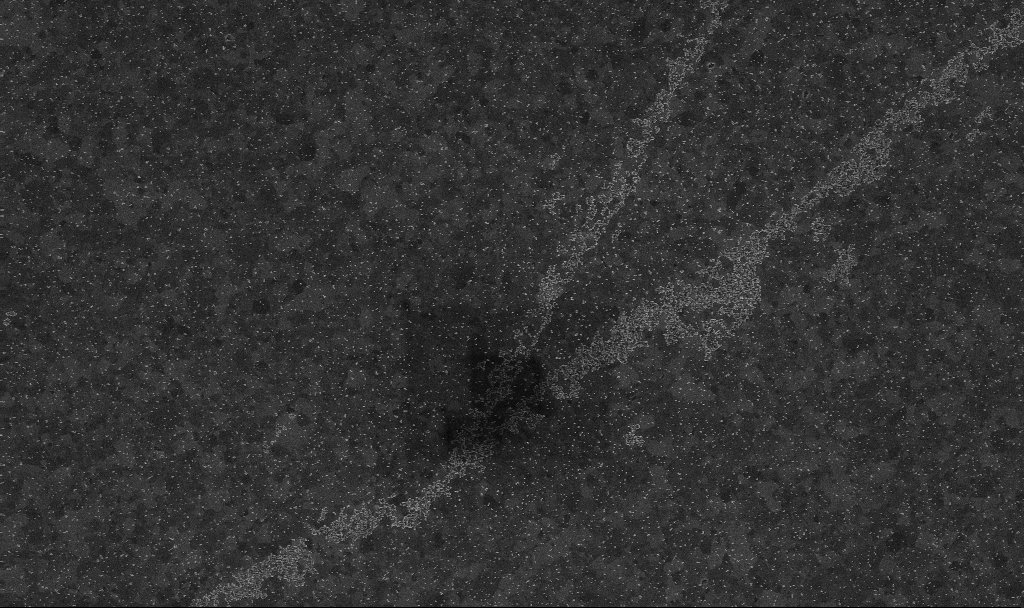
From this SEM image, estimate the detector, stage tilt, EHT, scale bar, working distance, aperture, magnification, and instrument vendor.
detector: InLens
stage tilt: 0°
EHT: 10 kV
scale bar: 1000 nm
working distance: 3.4 mm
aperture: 30 µm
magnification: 20 K X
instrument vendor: Zeiss